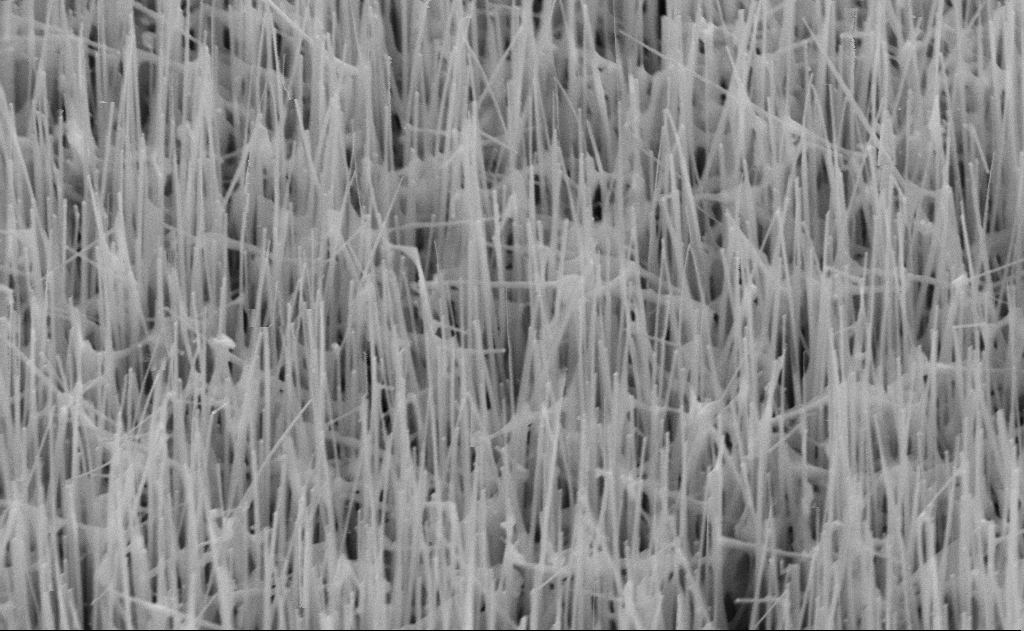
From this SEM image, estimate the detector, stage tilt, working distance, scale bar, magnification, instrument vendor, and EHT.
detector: SE2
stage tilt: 45°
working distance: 11 mm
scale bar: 1000 nm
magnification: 60 K X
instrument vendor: Zeiss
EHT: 10 kV